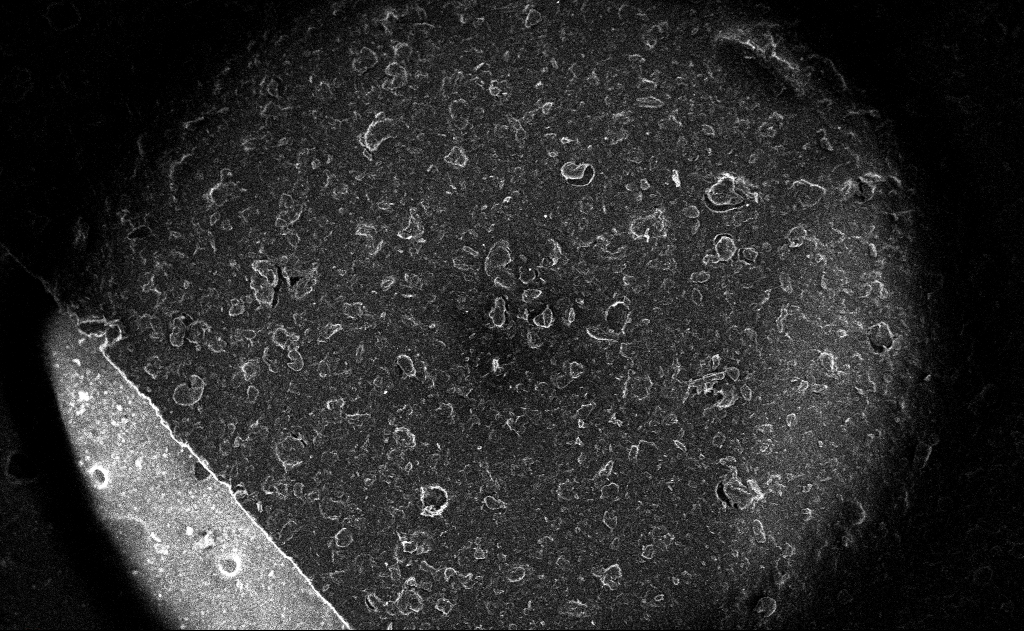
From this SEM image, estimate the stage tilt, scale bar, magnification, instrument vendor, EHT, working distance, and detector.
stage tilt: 0°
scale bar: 100000 nm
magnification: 0.122 K X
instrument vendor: Zeiss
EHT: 10 kV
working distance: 3 mm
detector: InLens